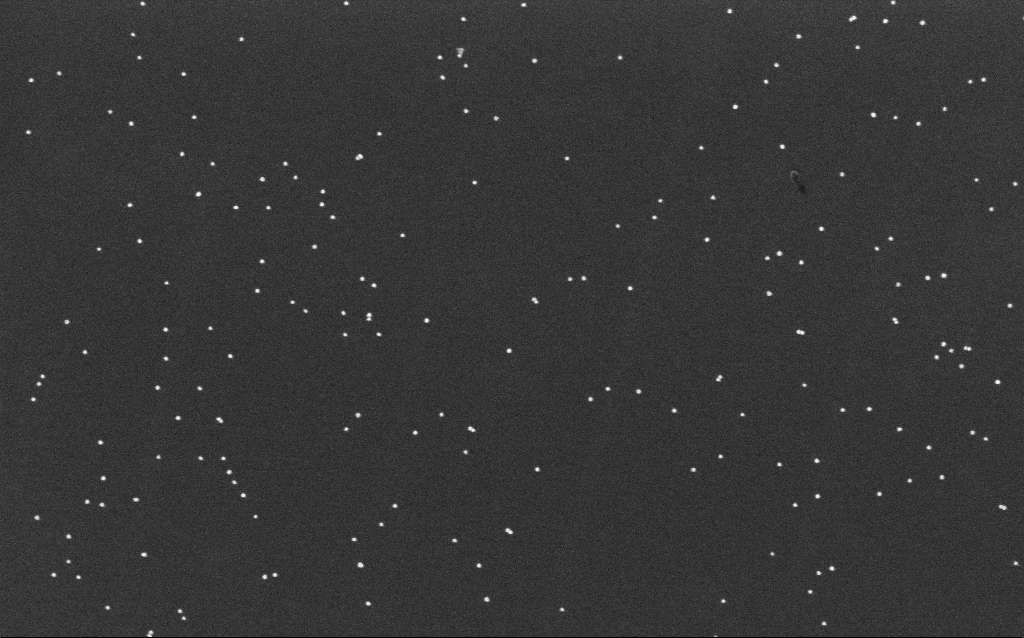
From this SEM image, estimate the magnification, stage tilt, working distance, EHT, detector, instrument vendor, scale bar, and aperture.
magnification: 100 K X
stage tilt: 0°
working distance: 6.4 mm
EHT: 10 kV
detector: InLens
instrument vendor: Zeiss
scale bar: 200 nm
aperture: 30 µm